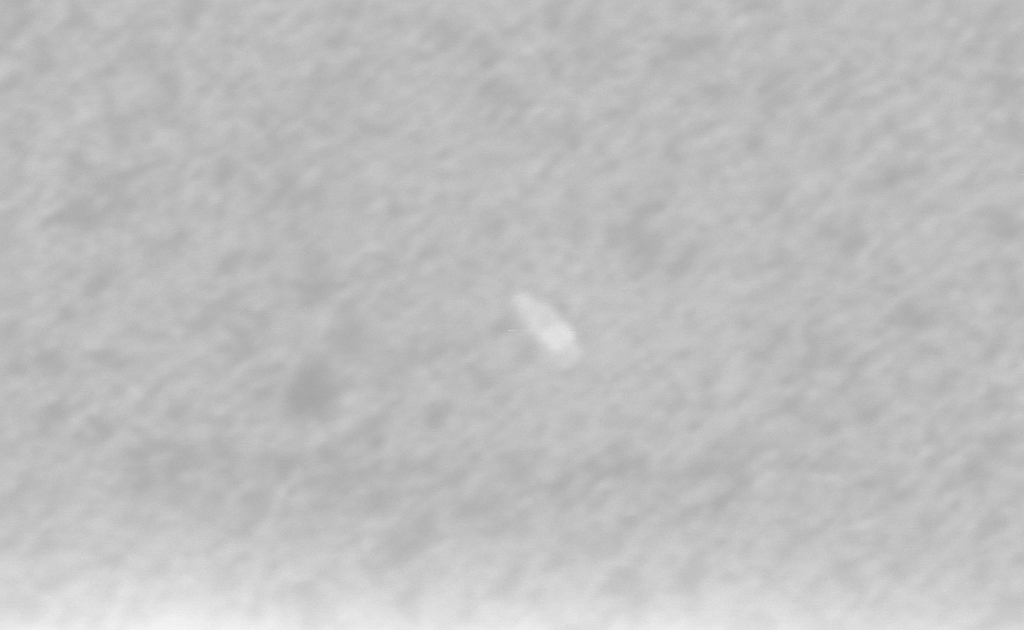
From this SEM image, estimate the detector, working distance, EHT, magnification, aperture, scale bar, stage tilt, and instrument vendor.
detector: InLens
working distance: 2.5 mm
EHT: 3 kV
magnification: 389.11 K X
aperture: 30 µm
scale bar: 100 nm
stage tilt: -0°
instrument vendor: Zeiss